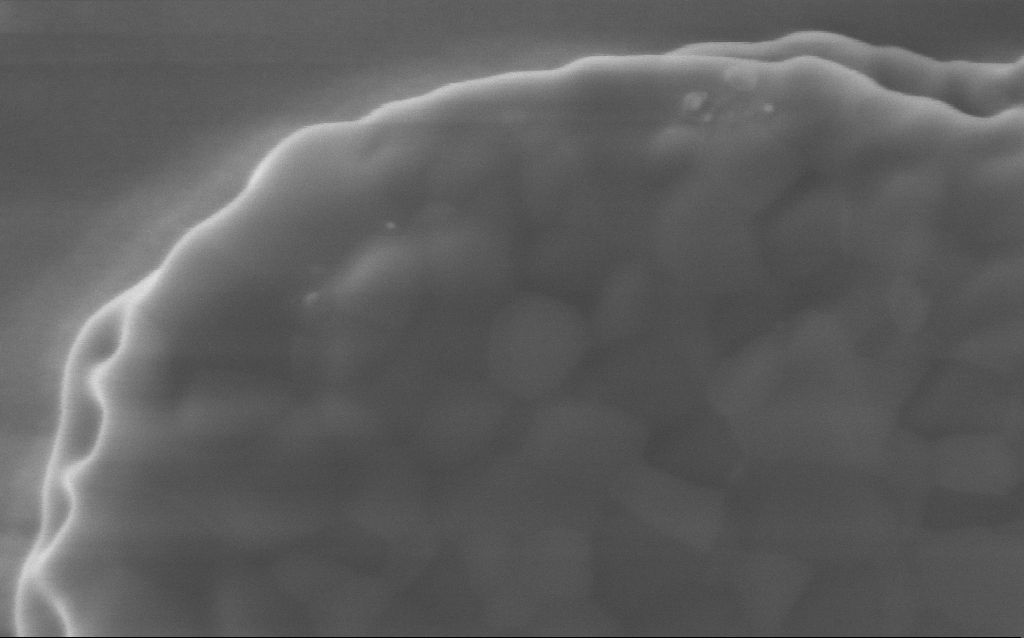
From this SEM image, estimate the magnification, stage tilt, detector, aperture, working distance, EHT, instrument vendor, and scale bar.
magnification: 172 K X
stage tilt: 0°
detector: InLens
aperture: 30 µm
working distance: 4 mm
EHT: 5 kV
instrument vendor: Zeiss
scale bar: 200 nm